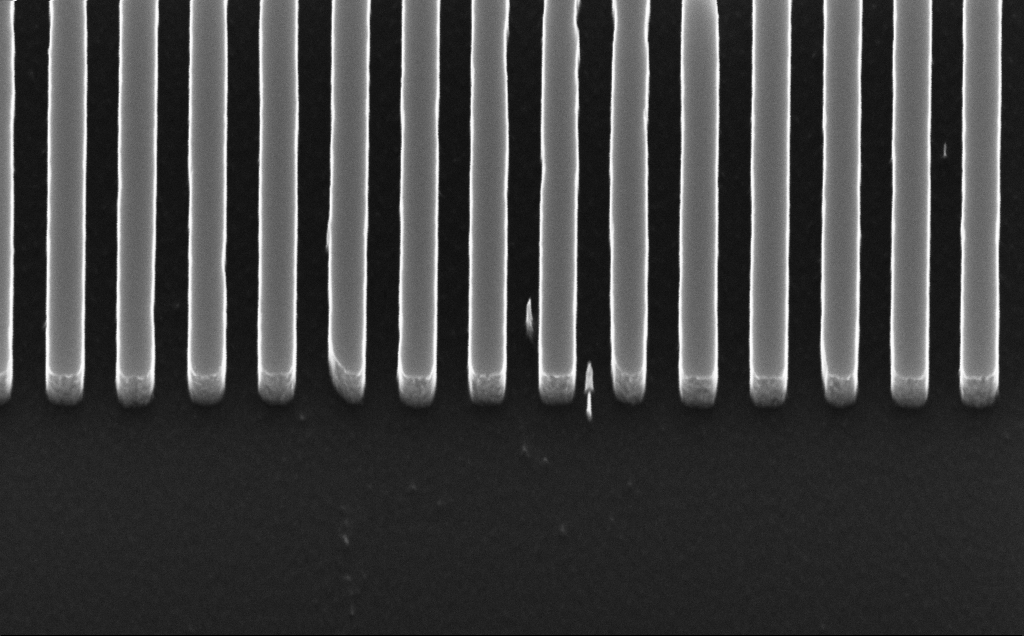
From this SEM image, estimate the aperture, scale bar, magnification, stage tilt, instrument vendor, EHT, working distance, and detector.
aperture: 30 µm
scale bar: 200 nm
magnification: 74.06 K X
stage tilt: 30°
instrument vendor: Zeiss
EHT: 10 kV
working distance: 5 mm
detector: InLens